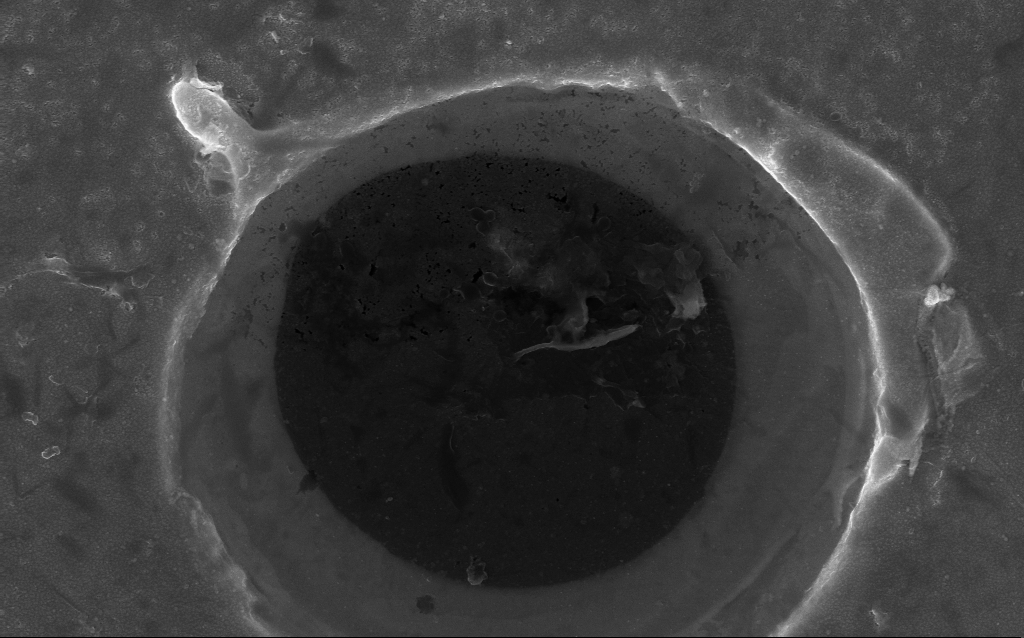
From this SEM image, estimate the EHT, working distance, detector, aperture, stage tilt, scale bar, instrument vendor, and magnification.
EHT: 10 kV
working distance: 4.6 mm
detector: InLens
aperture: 30 µm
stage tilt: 0°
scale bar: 1000 nm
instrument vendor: Zeiss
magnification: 43.63 K X